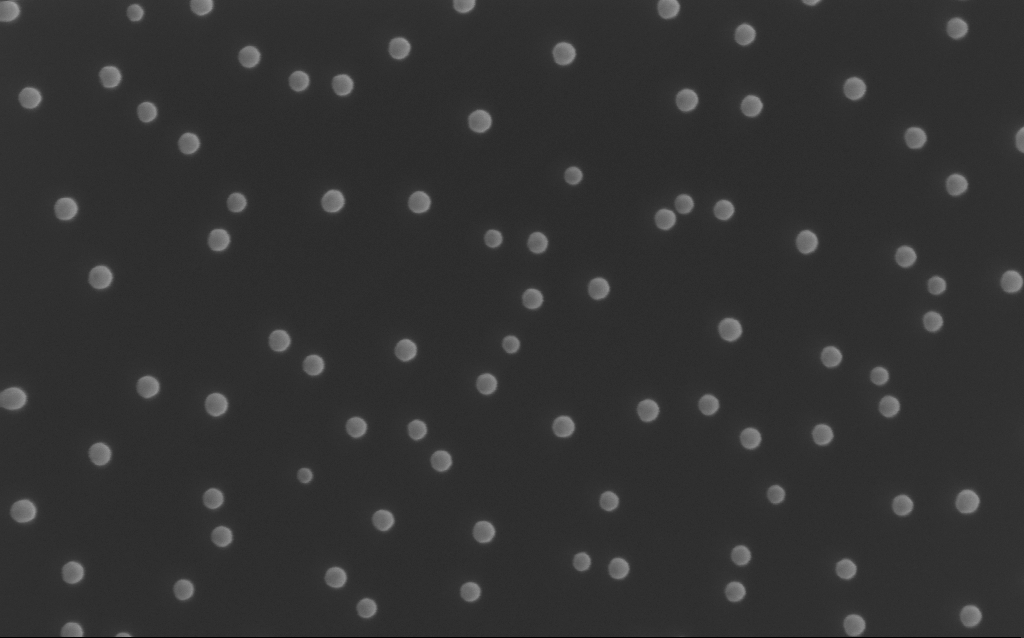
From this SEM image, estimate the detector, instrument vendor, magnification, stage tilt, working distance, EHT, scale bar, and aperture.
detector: InLens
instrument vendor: Zeiss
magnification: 142.09 K X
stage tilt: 0°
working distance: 5 mm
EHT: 10 kV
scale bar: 200 nm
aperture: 30 µm